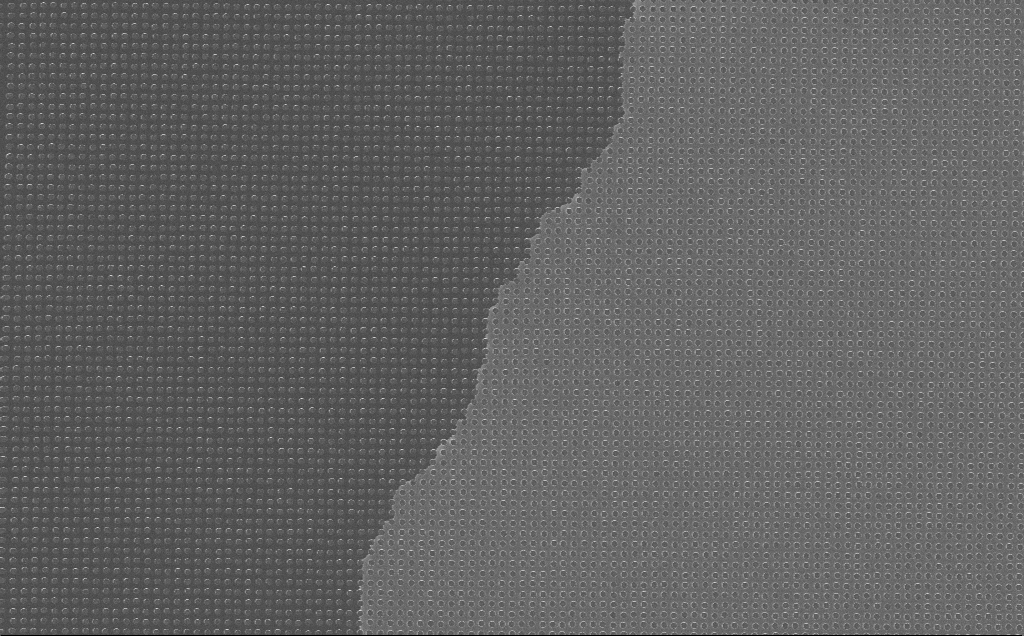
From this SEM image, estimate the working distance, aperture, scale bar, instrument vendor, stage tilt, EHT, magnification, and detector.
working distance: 7 mm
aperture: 30 µm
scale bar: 2000 nm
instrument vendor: Zeiss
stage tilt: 0°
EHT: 10 kV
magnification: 9.36 K X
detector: InLens